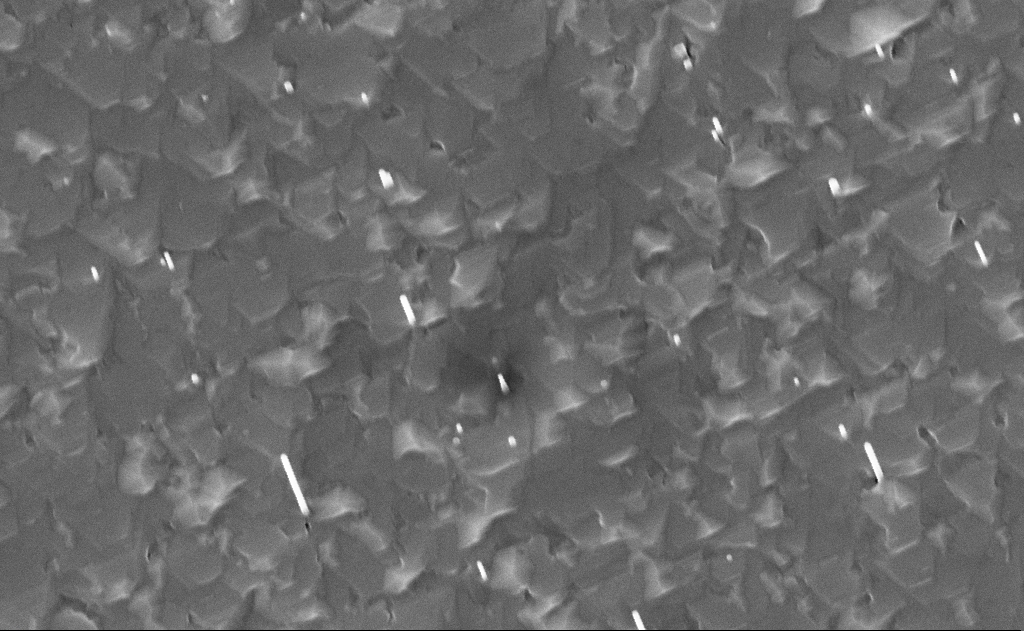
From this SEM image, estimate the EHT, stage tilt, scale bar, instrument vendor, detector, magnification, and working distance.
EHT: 10 kV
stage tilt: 0°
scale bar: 1000 nm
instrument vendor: Zeiss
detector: InLens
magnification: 50 K X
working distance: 14 mm